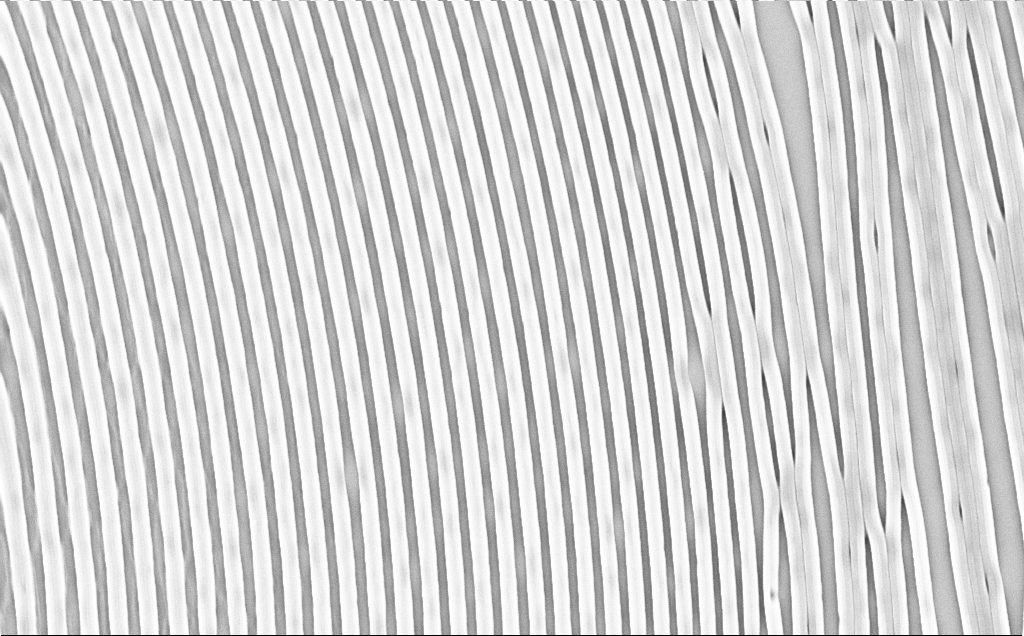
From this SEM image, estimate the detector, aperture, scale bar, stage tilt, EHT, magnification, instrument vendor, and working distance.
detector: InLens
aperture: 30 µm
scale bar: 2000 nm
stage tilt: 0°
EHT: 5 kV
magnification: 20.48 K X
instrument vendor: Zeiss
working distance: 6 mm